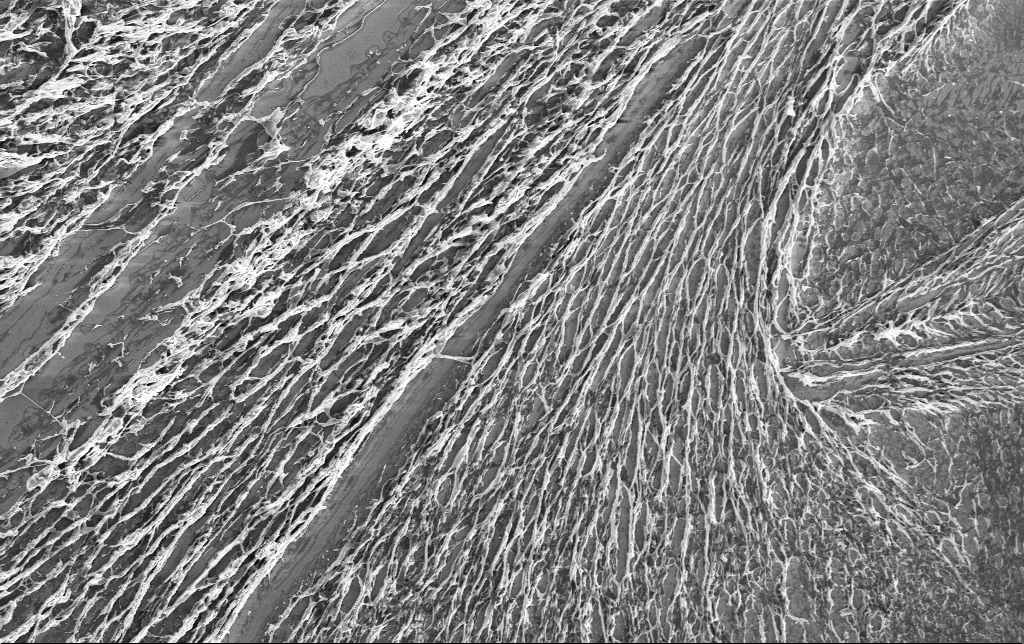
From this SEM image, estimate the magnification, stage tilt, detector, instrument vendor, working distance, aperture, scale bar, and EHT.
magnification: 2.48 K X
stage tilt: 0°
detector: InLens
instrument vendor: Zeiss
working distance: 3.2 mm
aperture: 30 µm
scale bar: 10000 nm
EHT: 3 kV